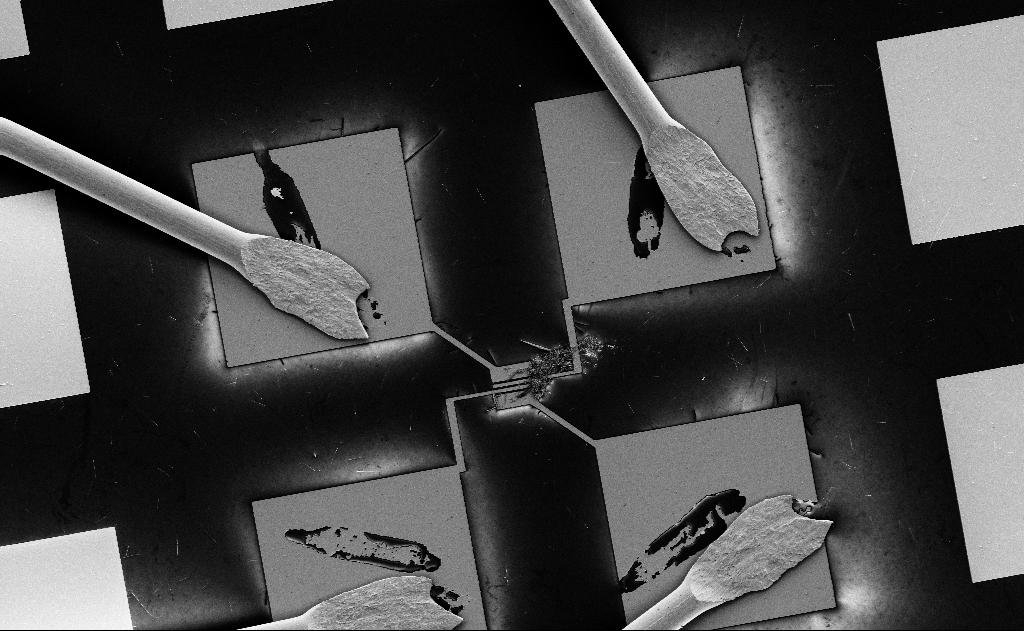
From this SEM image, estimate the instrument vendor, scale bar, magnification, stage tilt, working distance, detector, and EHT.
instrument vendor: Zeiss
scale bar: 100000 nm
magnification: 0.507 K X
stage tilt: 0°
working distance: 18 mm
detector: SE2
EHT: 5 kV